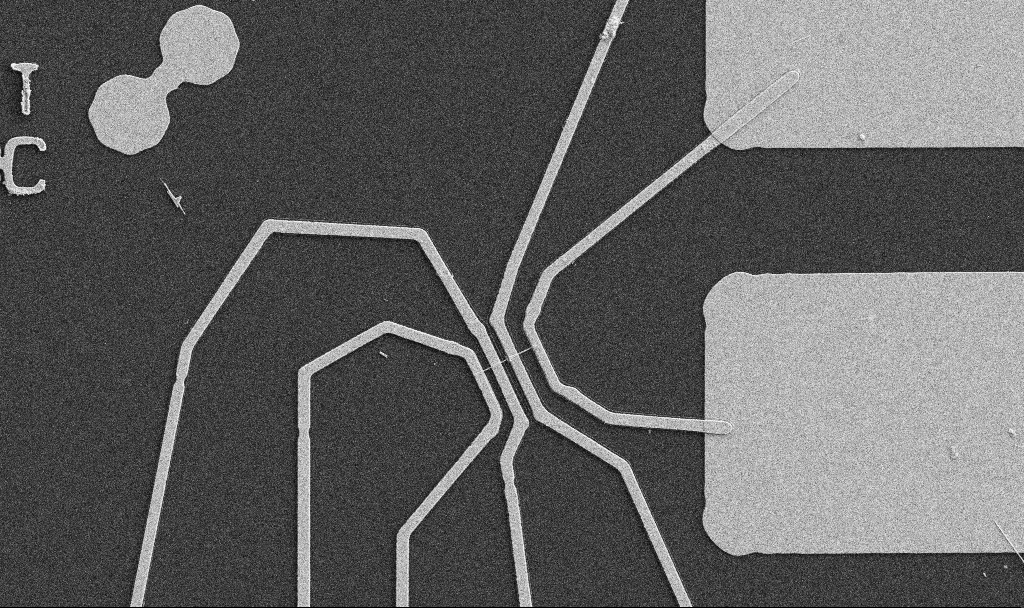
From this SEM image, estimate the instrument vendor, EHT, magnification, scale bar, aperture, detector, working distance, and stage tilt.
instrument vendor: Zeiss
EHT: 5 kV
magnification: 5 K X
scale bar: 10000 nm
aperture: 30 µm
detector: SE2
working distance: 10.7 mm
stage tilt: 0°